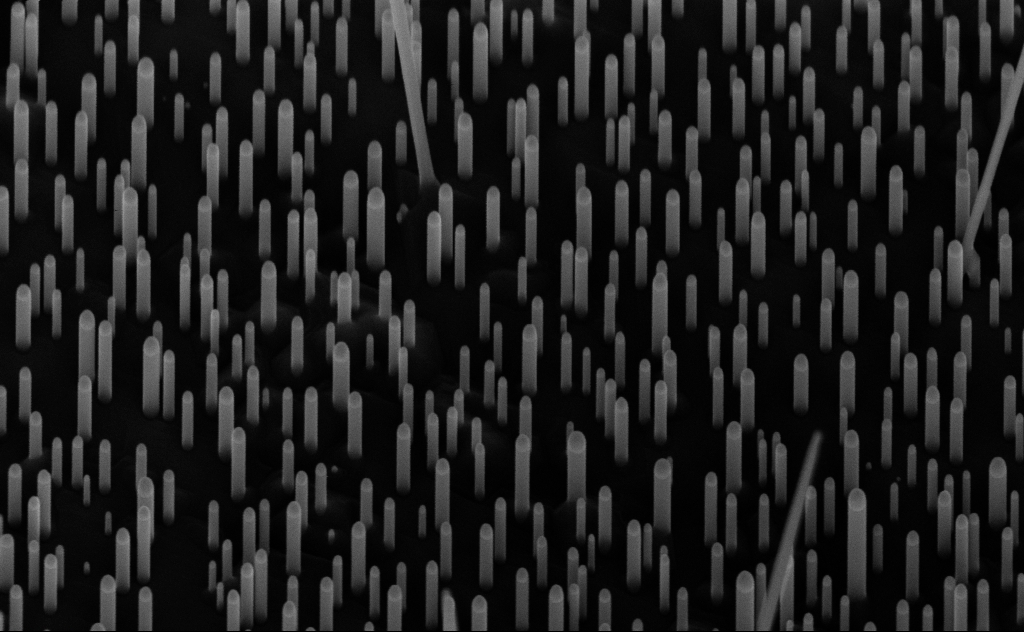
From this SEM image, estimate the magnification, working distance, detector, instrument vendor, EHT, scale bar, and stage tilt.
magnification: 80 K X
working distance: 7 mm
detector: InLens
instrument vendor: Zeiss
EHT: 10 kV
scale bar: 200 nm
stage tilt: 45°